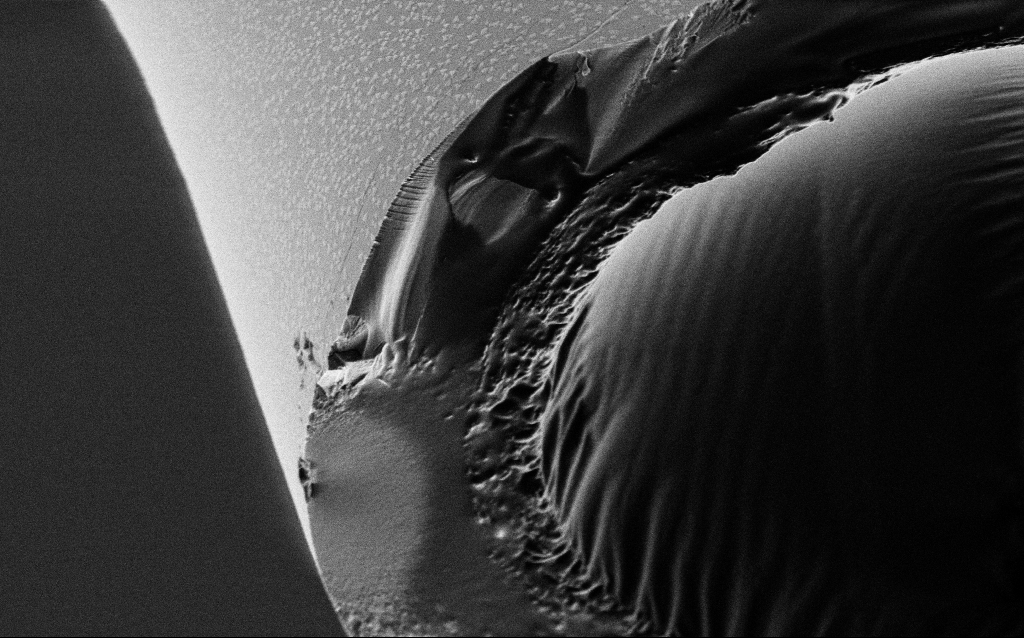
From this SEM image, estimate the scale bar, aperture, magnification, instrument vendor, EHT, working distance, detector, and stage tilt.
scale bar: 2000 nm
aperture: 30 µm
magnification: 25 K X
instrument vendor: Zeiss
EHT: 1 kV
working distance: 6.6 mm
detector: SE2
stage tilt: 45°